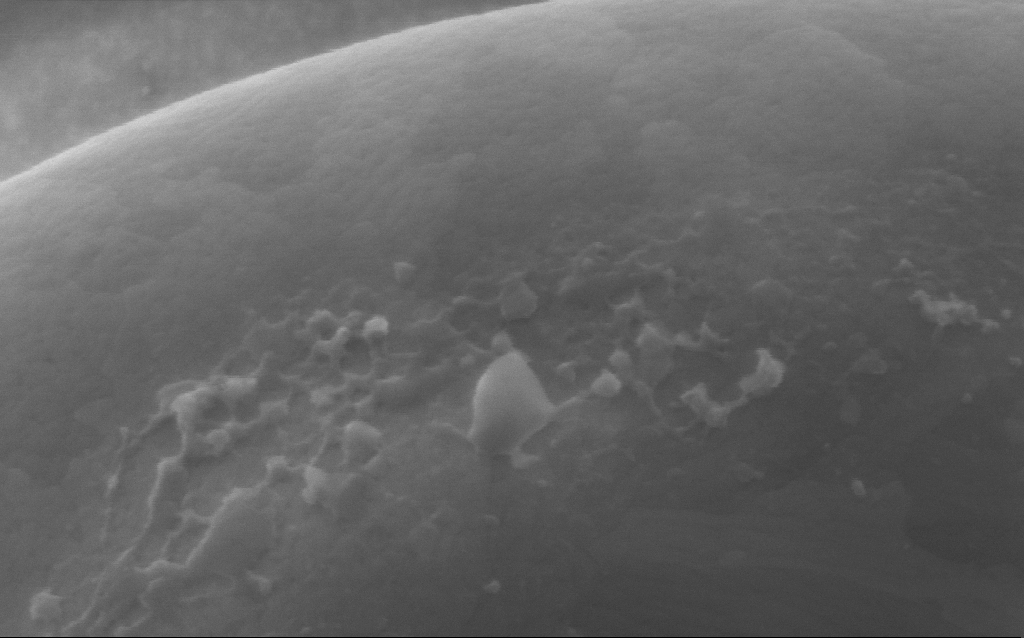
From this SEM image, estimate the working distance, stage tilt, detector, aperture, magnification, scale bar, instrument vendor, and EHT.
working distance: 4 mm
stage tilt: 0°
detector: InLens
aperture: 30 µm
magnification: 254 K X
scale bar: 200 nm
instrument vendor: Zeiss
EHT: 5 kV